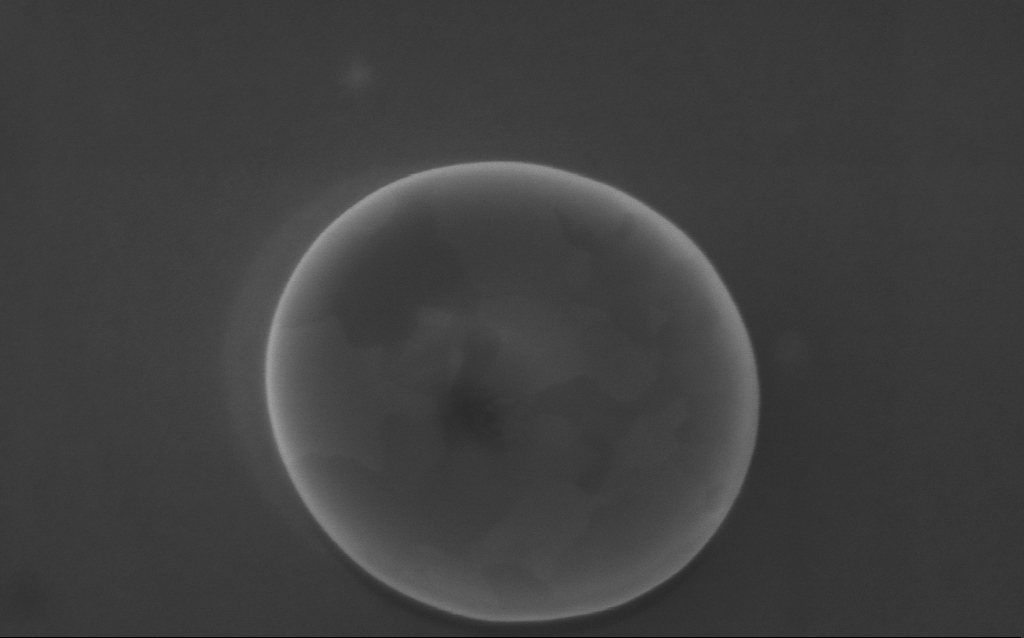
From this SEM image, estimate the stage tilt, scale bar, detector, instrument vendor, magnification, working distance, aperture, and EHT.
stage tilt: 0°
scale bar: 200 nm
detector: InLens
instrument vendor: Zeiss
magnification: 105 K X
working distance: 3 mm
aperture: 30 µm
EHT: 5 kV